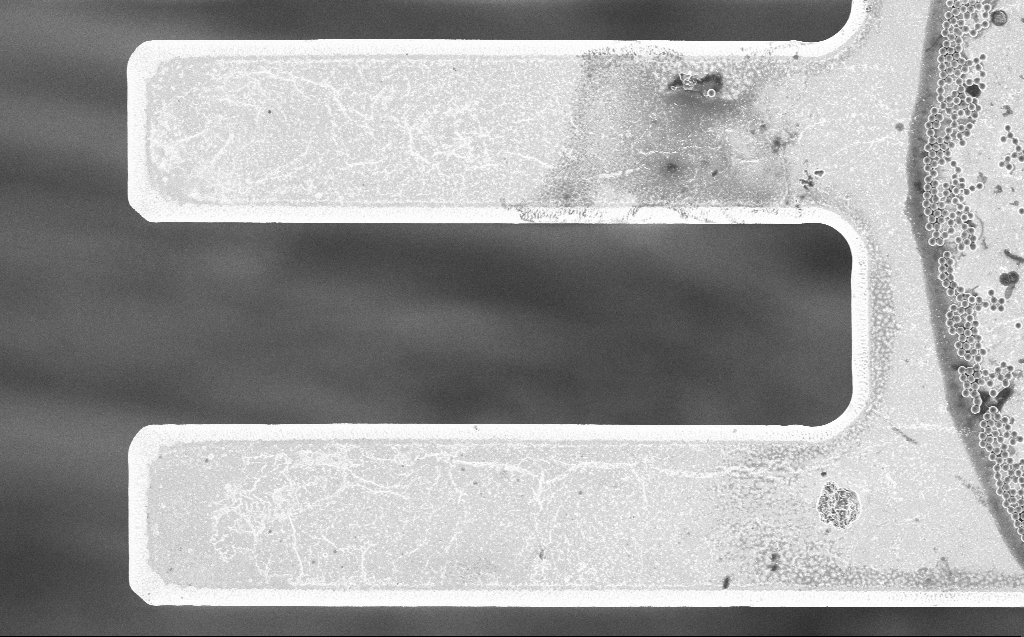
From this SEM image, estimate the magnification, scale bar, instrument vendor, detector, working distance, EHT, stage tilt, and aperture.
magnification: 3.54 K X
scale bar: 20000 nm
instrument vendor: Zeiss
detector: InLens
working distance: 7 mm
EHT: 3 kV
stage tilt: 0°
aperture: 30 µm